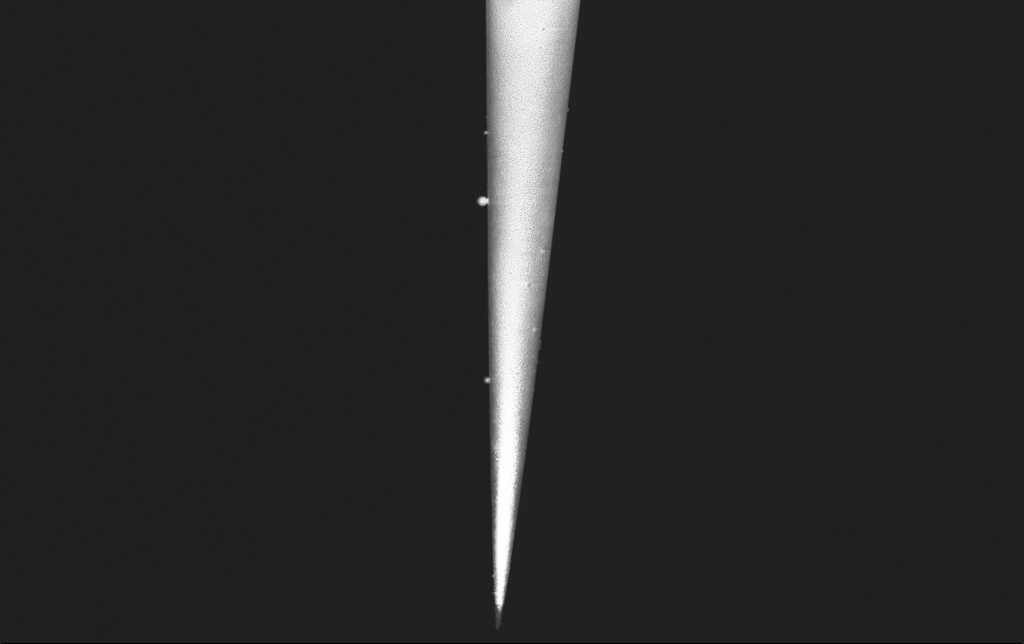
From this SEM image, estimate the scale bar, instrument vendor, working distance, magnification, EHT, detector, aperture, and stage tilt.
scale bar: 2000 nm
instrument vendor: Zeiss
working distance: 5.9 mm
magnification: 10 K X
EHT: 2 kV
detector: InLens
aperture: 30 µm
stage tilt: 0°